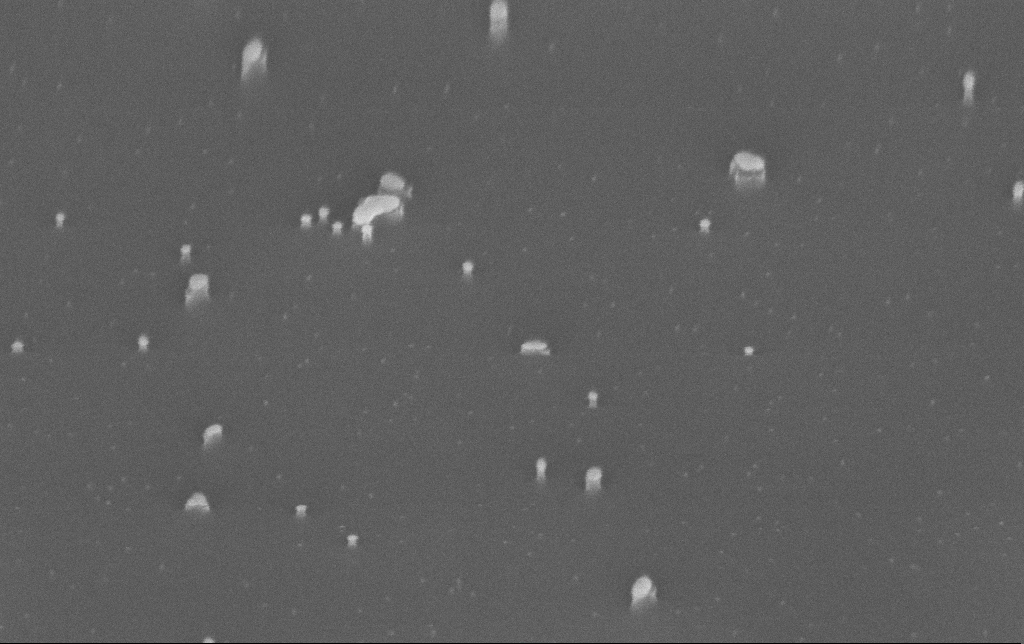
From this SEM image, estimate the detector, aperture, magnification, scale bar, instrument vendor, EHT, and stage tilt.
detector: InLens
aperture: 30 µm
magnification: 200 K X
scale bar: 100 nm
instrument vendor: Zeiss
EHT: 10 kV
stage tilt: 45°